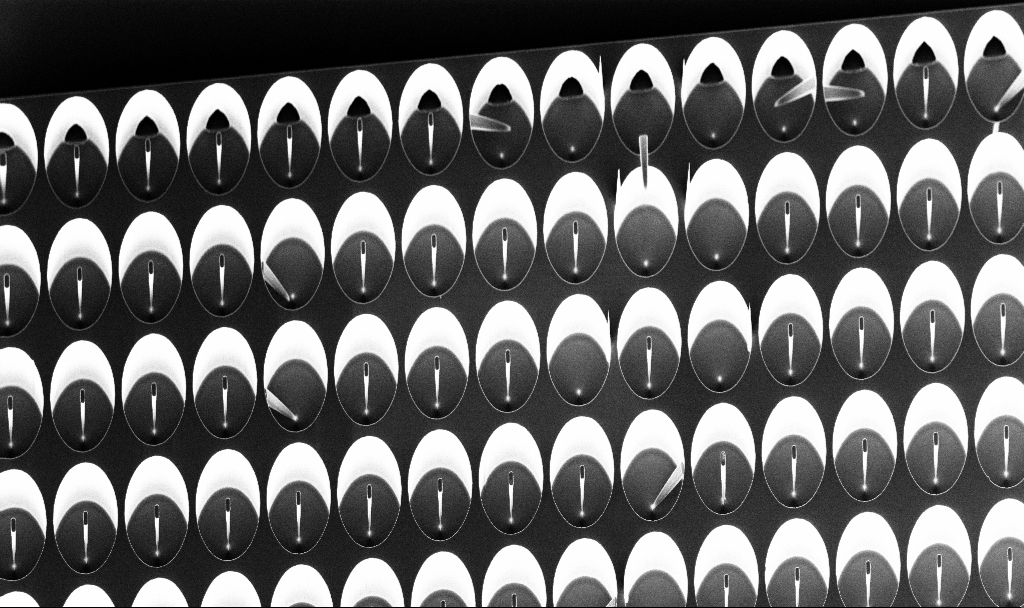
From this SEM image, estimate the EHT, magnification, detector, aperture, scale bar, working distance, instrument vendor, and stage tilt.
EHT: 5 kV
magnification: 0.791 K X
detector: InLens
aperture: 30 µm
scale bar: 20000 nm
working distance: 6.8 mm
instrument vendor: Zeiss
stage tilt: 29.2°